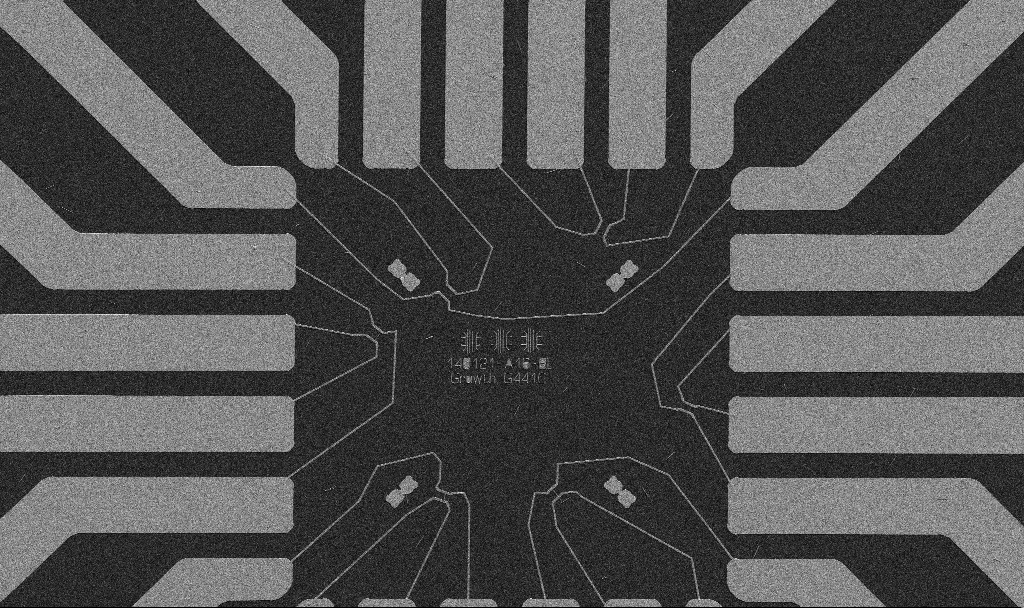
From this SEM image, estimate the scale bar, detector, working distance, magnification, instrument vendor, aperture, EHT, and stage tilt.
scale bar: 20000 nm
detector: SE2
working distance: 10.7 mm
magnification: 1 K X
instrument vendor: Zeiss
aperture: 30 µm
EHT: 5 kV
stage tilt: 0°